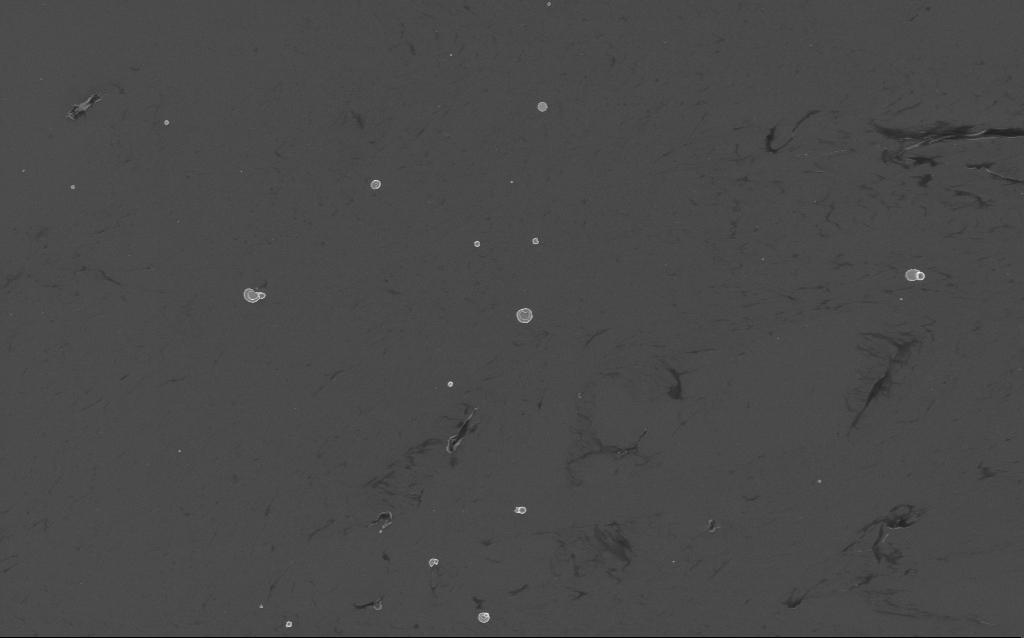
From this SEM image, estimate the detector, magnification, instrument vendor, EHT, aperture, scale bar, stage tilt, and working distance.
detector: InLens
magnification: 3.64 K X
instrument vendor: Zeiss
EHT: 3 kV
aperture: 30 µm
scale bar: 10000 nm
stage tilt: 0°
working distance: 3 mm